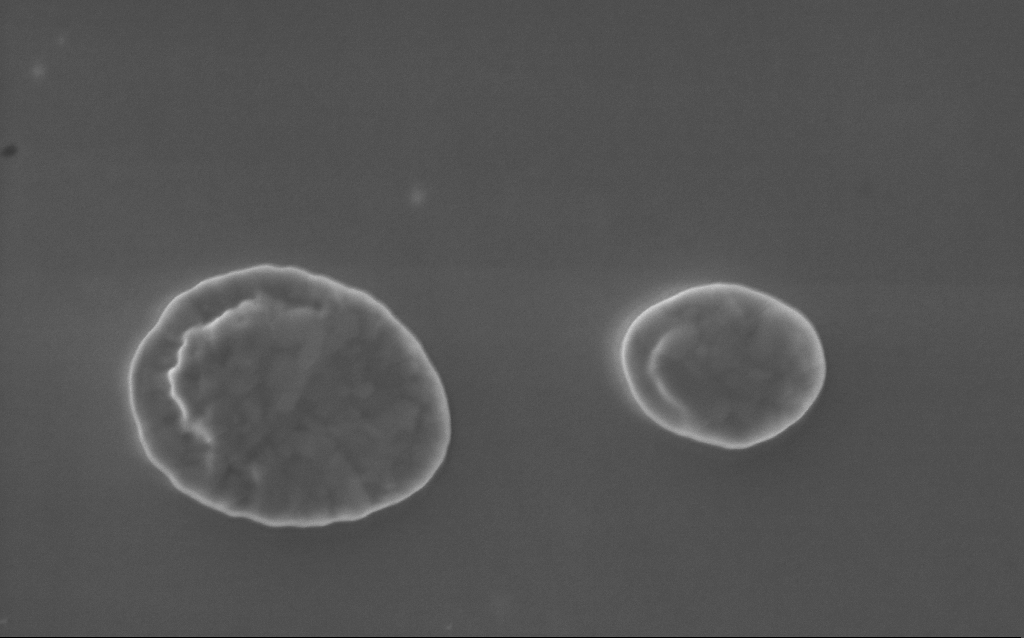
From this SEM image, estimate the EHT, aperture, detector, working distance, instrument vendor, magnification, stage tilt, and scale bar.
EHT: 5 kV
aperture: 30 µm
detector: InLens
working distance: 3 mm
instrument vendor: Zeiss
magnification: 58 K X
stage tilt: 0°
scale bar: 1000 nm